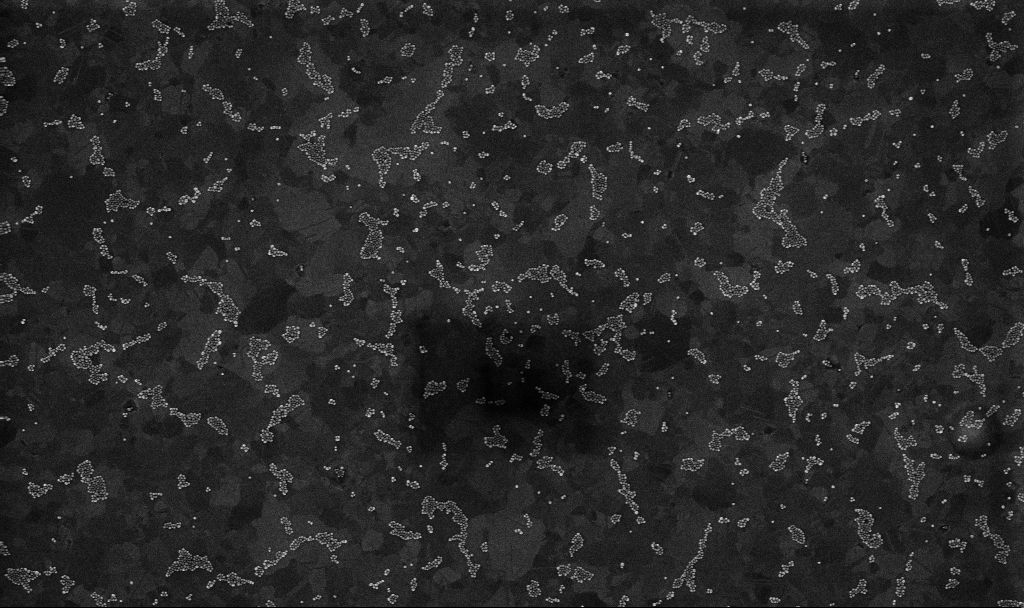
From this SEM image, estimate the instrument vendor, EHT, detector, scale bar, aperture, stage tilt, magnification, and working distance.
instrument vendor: Zeiss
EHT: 10 kV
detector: InLens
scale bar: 1000 nm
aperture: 30 µm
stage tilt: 0°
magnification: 50 K X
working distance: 3.4 mm